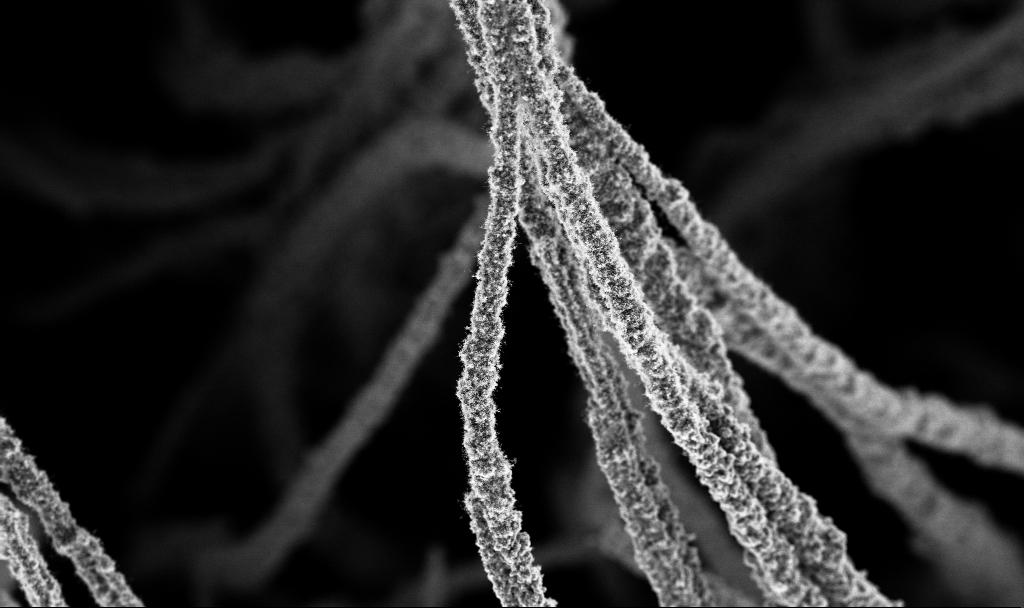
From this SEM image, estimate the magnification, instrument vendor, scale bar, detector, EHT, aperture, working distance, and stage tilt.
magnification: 1 K X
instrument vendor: Zeiss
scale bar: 20000 nm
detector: InLens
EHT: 3 kV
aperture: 30 µm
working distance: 2.7 mm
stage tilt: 0°